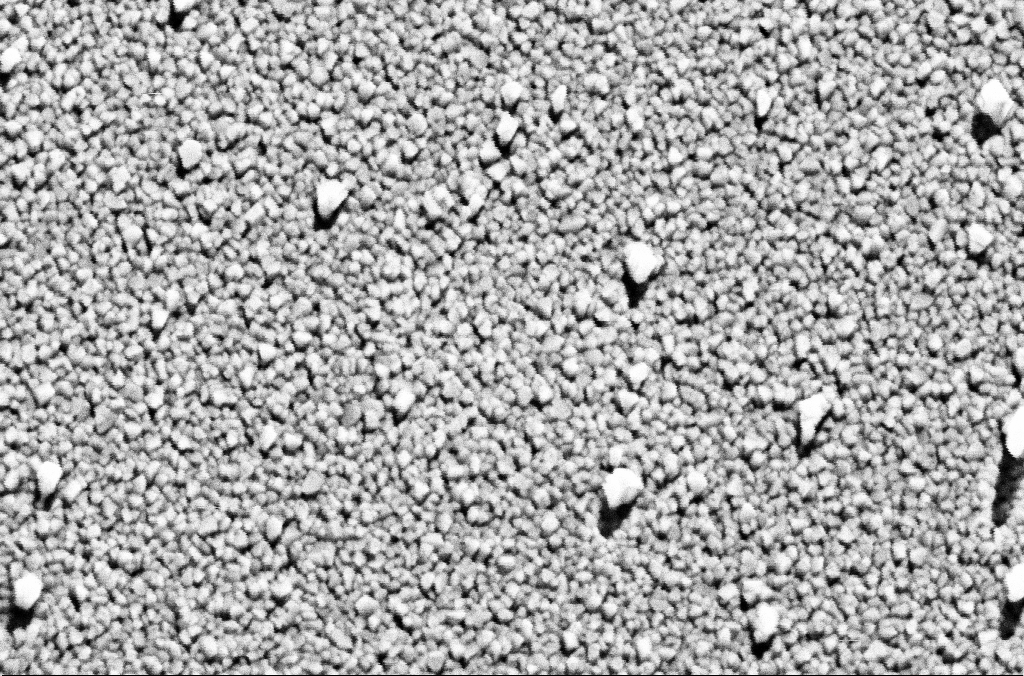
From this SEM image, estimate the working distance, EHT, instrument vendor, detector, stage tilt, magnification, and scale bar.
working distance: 8.5 mm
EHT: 5 kV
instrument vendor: Zeiss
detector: SE2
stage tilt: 0°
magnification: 165.9 K X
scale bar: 200 nm